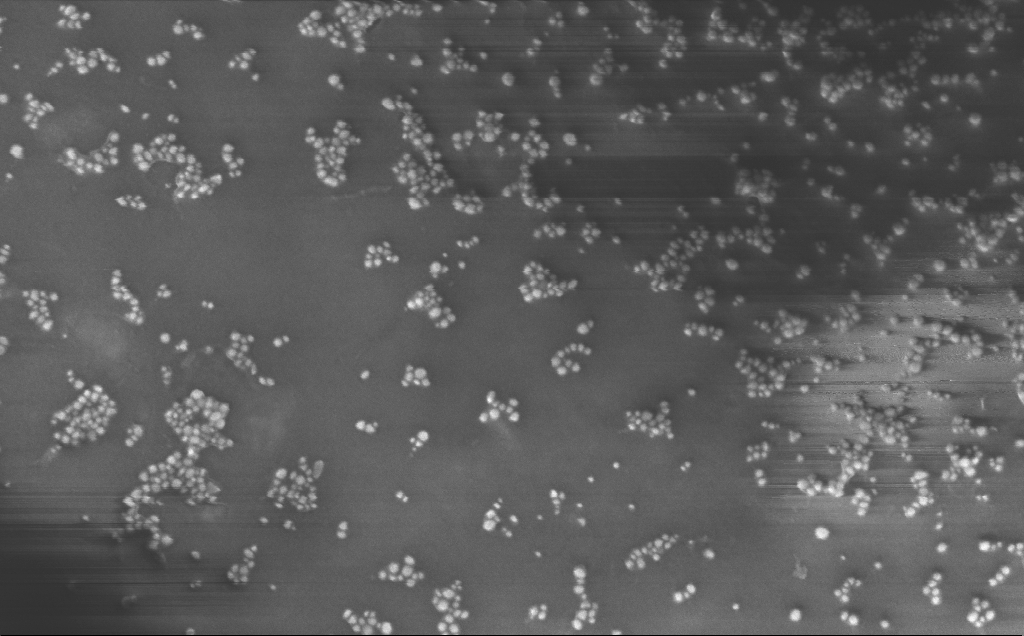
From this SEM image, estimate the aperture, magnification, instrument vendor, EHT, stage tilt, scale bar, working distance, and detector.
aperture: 30 µm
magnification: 146.17 K X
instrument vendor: Zeiss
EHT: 10 kV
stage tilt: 0°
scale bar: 200 nm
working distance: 4 mm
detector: InLens